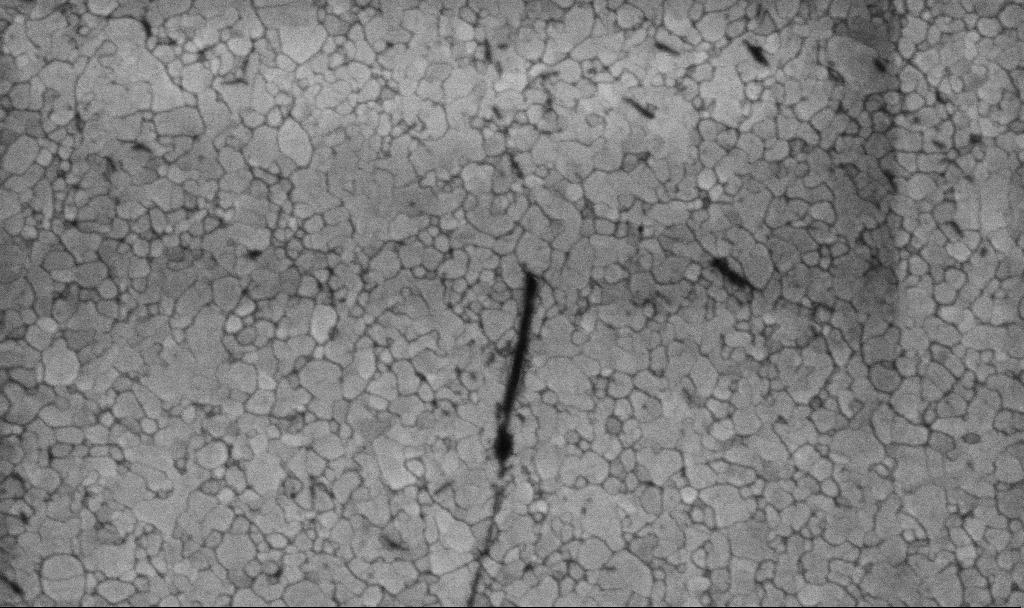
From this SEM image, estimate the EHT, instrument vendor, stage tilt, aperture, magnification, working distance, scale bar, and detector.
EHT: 5 kV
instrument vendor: Zeiss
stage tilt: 0°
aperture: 30 µm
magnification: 100.15 K X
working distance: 3.1 mm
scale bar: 200 nm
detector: InLens